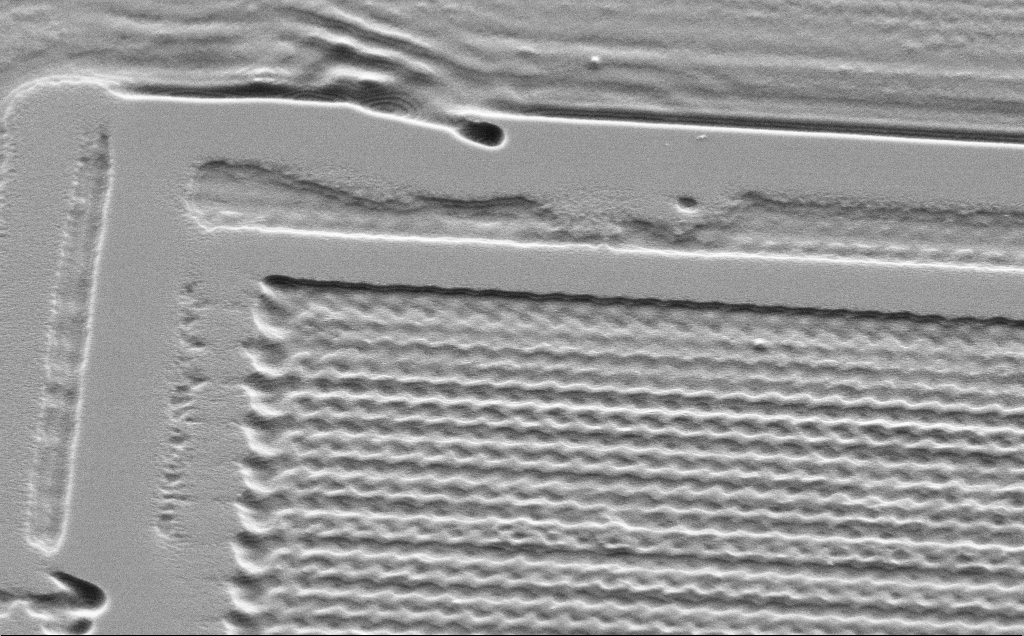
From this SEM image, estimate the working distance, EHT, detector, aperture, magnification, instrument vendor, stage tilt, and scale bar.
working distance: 10 mm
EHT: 5 kV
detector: SE2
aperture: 30 µm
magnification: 5.83 K X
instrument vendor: Zeiss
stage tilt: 45°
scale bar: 10000 nm